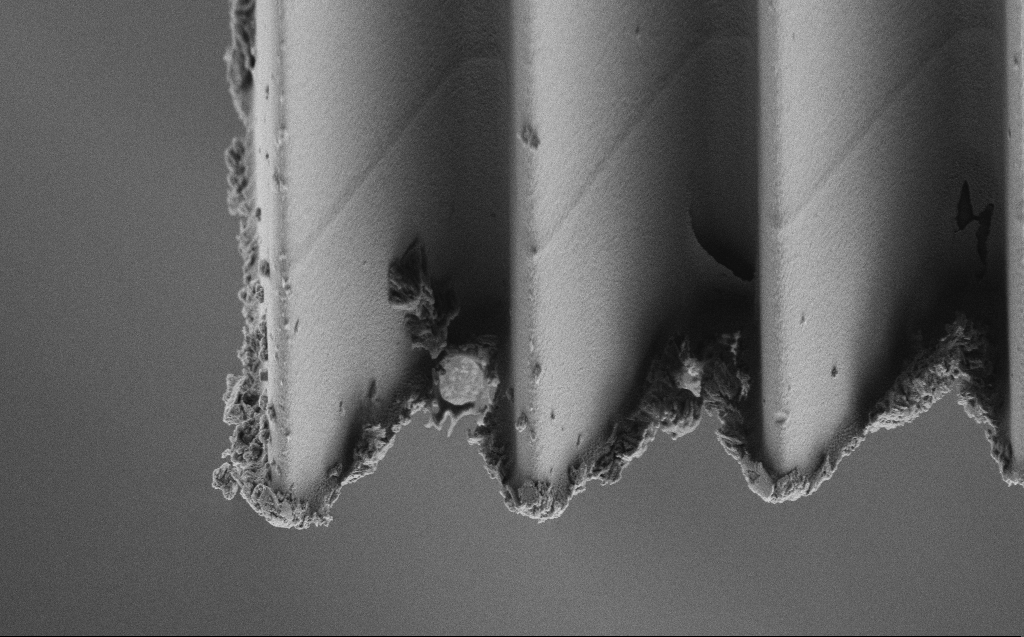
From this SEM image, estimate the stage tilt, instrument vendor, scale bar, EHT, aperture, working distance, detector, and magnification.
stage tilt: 45°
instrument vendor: Zeiss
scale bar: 2000 nm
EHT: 3 kV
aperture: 30 µm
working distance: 4 mm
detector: SE2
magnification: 8.06 K X